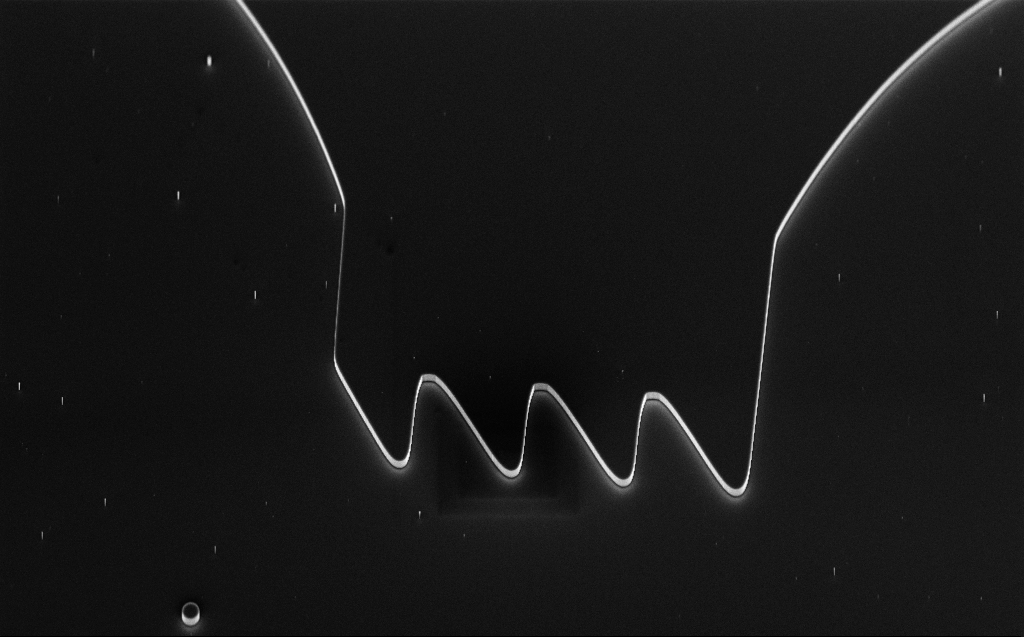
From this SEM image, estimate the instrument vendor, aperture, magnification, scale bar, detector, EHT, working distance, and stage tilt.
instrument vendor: Zeiss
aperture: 30 µm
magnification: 3.67 K X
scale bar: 10000 nm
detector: InLens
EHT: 5 kV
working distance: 5 mm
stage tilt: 45°